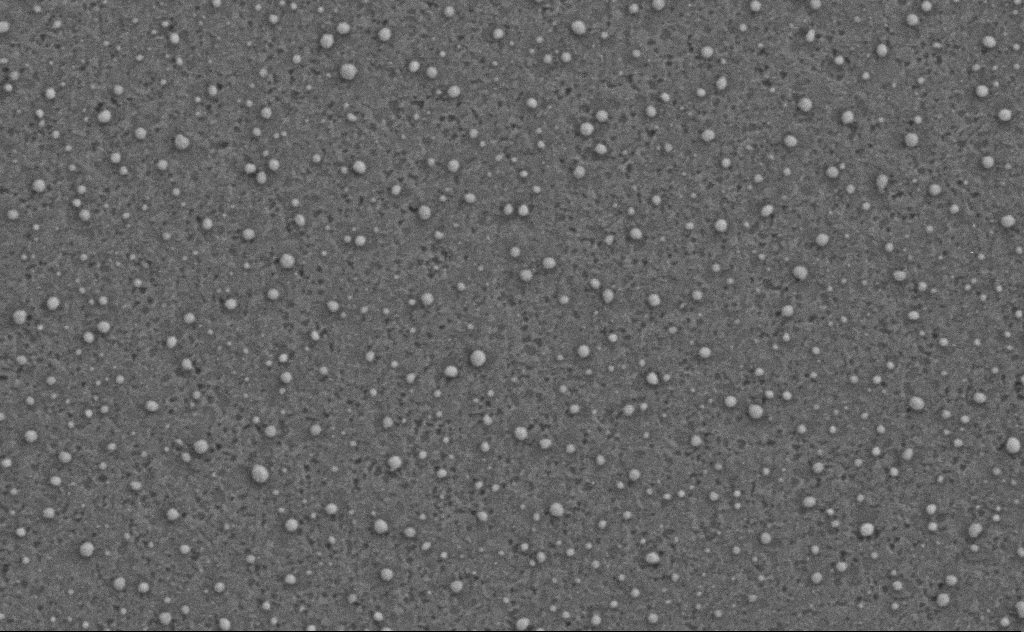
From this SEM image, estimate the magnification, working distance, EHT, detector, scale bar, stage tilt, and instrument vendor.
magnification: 80 K X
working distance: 4 mm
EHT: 3 kV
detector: SE2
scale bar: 200 nm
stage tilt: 0°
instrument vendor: Zeiss